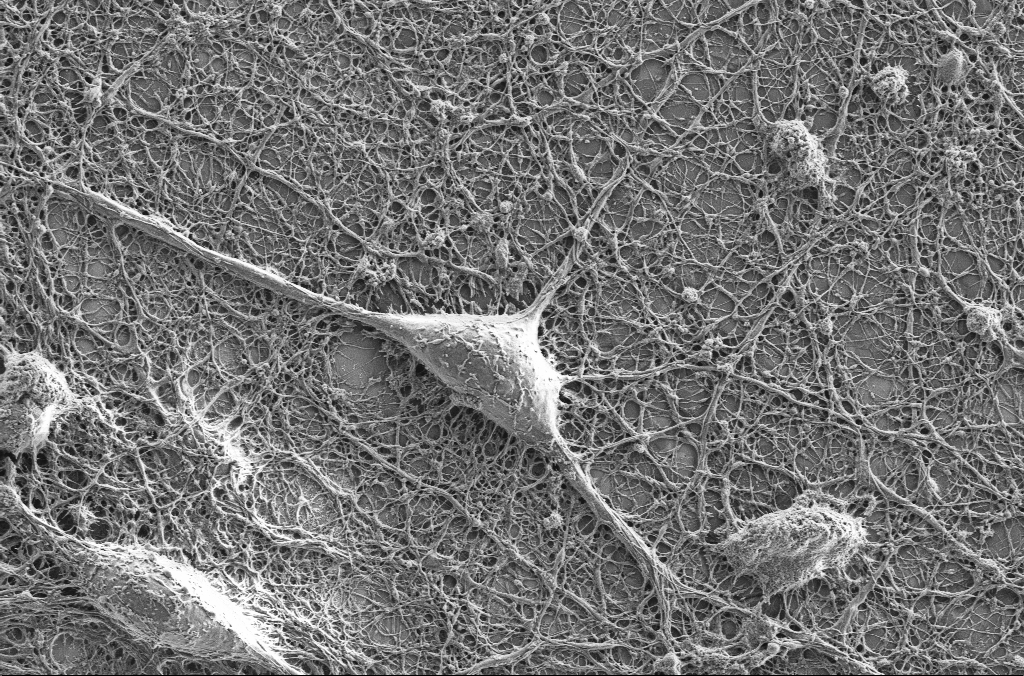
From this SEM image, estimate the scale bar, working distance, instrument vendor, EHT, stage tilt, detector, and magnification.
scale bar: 10000 nm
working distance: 4.1 mm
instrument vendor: Zeiss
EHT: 2 kV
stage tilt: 0°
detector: SE2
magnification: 5 K X